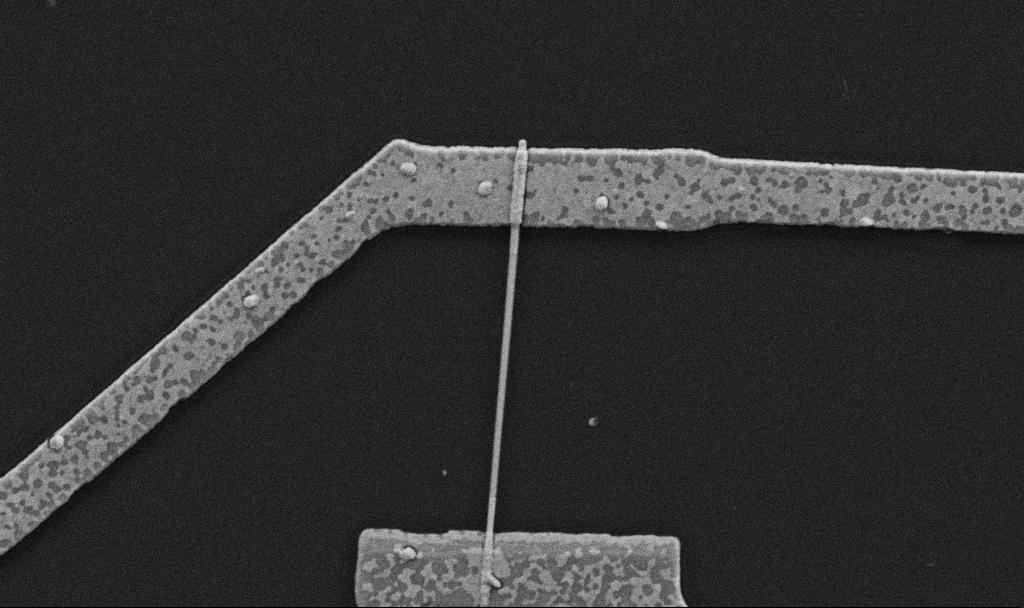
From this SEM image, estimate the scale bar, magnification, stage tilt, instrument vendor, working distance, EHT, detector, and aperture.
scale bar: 1000 nm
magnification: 30 K X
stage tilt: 0°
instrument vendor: Zeiss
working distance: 10.7 mm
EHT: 5 kV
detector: SE2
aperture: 30 µm